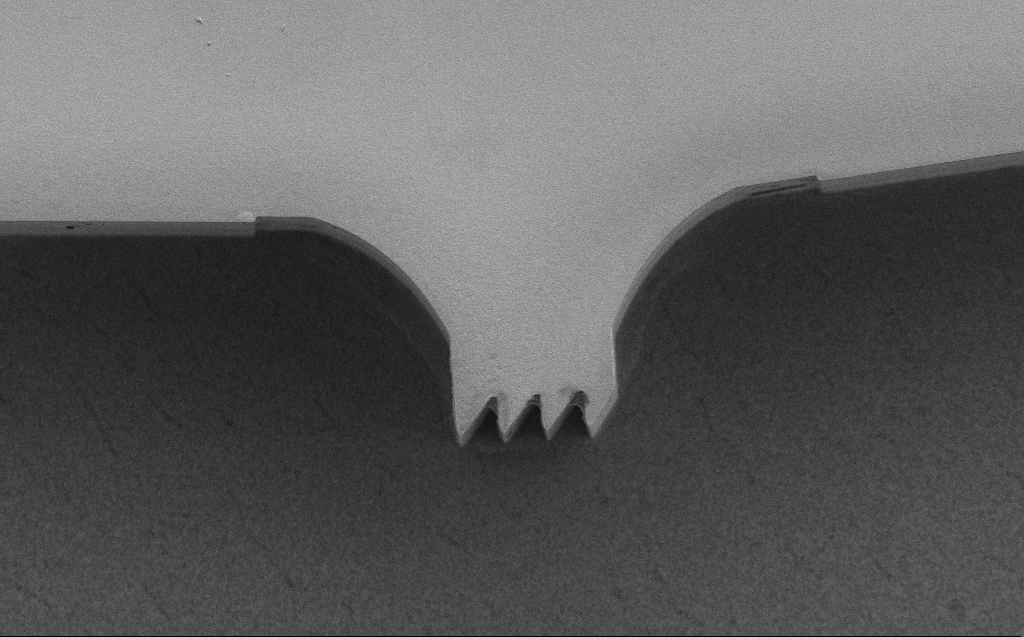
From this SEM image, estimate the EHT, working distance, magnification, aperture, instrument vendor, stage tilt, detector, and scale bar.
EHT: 5 kV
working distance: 7 mm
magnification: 1.4 K X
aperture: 30 µm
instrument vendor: Zeiss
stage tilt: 0°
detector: SE2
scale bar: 10000 nm